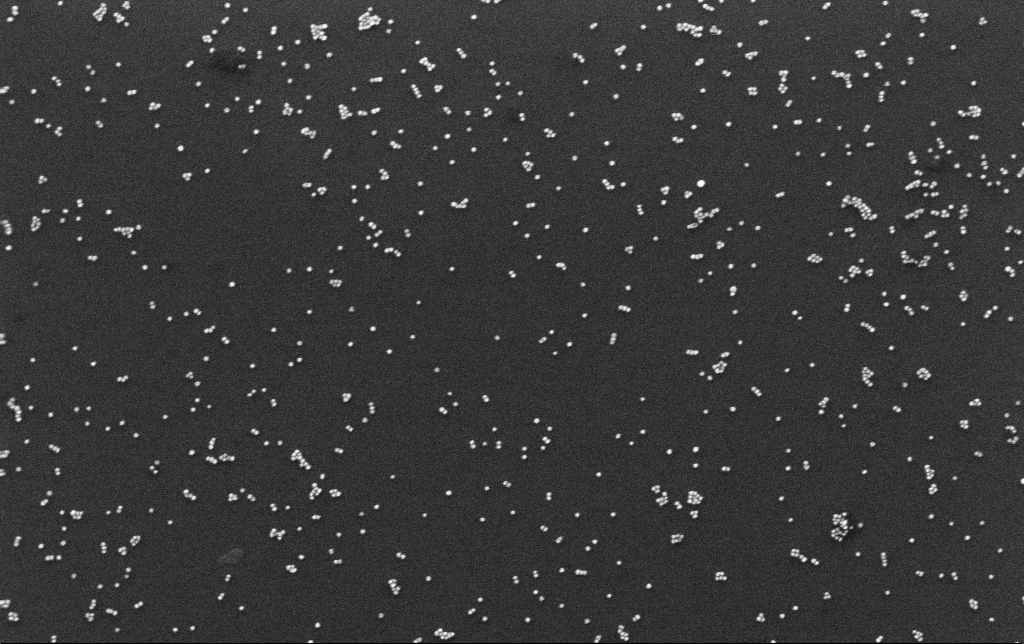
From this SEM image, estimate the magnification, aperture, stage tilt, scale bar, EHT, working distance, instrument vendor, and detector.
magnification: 100 K X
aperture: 30 µm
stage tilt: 0°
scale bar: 200 nm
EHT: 10 kV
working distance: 3 mm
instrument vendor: Zeiss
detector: InLens